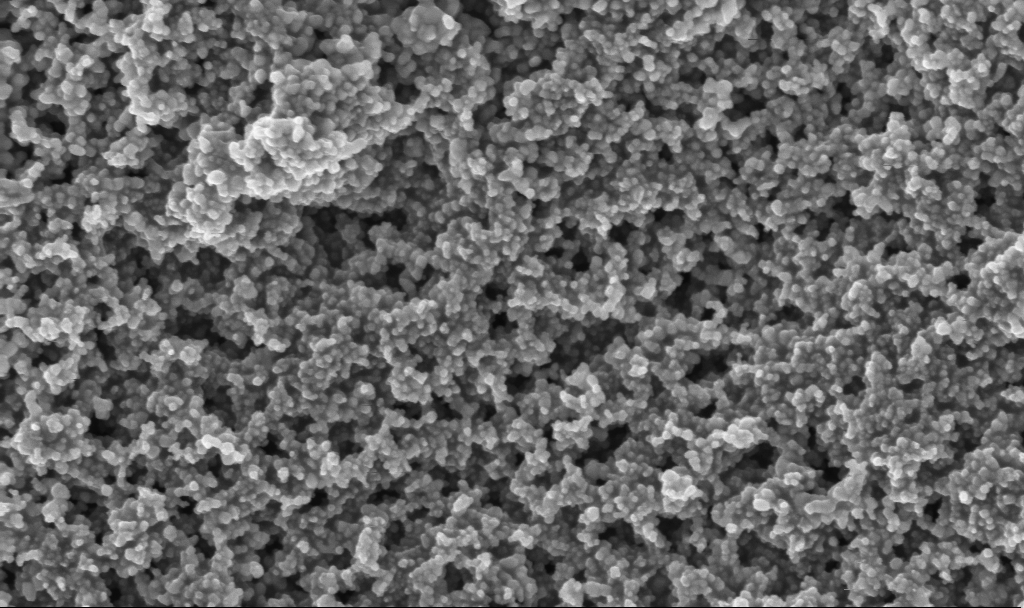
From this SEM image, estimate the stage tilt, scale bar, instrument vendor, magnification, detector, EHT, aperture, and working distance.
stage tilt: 0°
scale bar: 200 nm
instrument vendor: Zeiss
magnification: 100 K X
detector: InLens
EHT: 3 kV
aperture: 30 µm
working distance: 2.4 mm